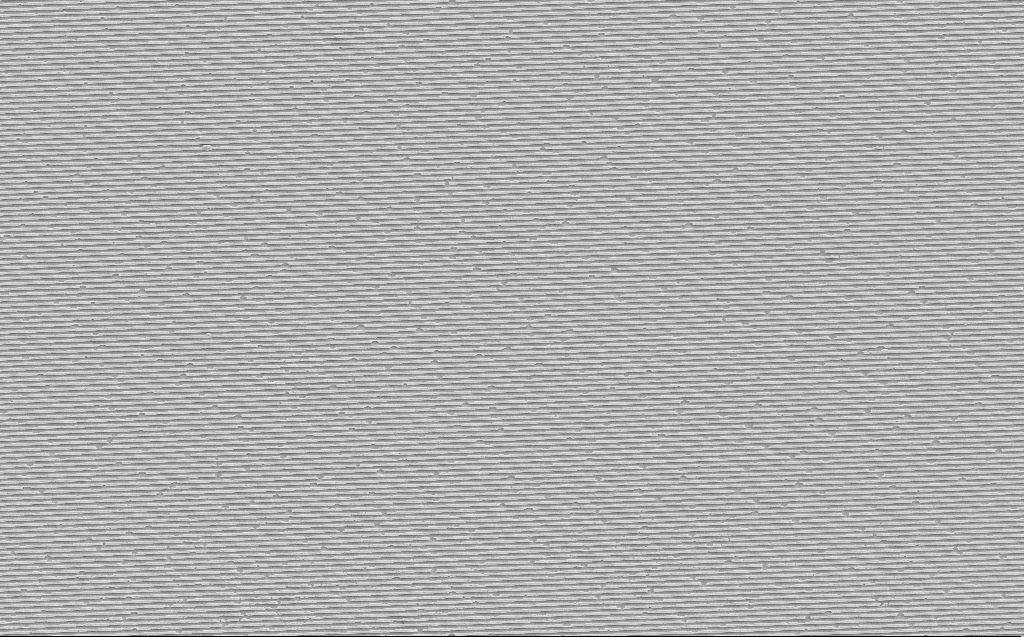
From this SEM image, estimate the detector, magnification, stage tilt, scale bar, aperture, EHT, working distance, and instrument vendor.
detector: SE2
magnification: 4 K X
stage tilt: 0°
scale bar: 10000 nm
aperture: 30 µm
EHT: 10 kV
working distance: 10 mm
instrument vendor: Zeiss